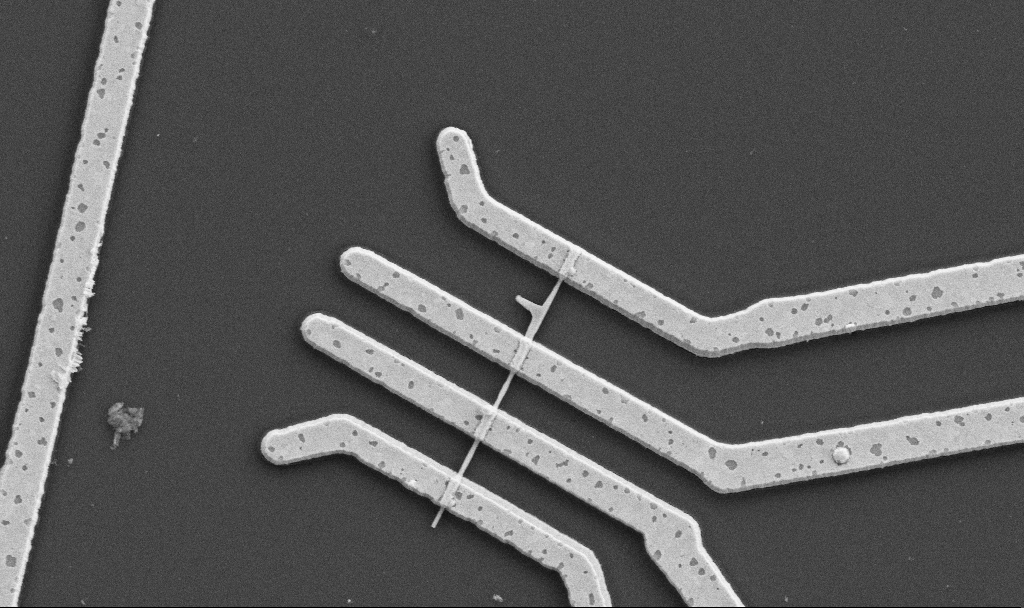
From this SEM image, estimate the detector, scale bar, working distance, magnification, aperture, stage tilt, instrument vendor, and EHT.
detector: SE2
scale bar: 1000 nm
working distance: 10.7 mm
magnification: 20 K X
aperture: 30 µm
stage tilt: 0°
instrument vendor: Zeiss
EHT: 5 kV